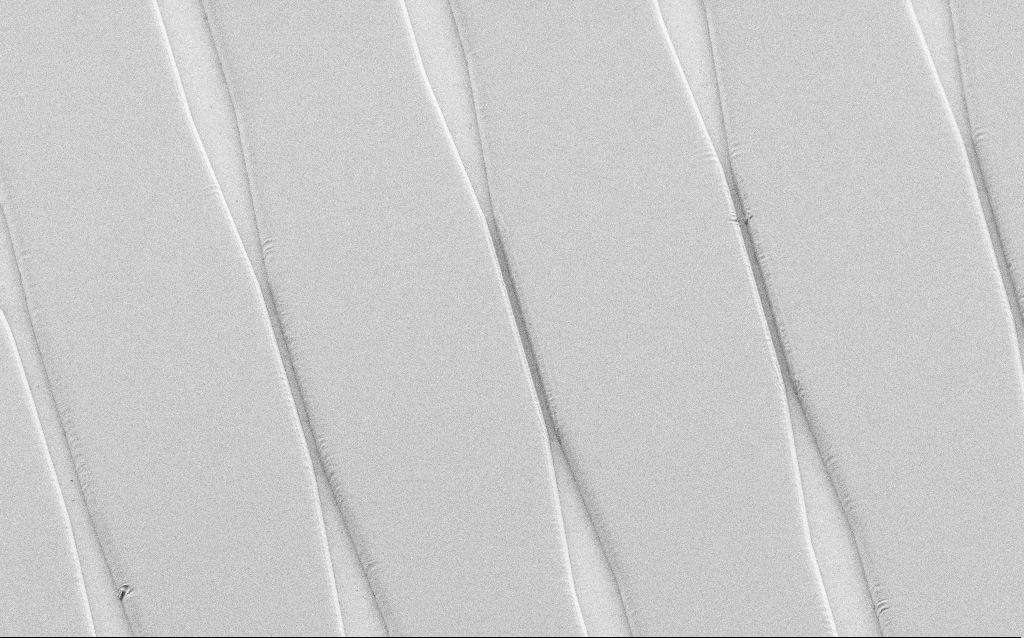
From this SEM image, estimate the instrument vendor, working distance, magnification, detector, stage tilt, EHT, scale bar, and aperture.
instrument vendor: Zeiss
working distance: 5 mm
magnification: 0.837 K X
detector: SE2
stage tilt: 36°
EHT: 1 kV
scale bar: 20000 nm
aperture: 30 µm